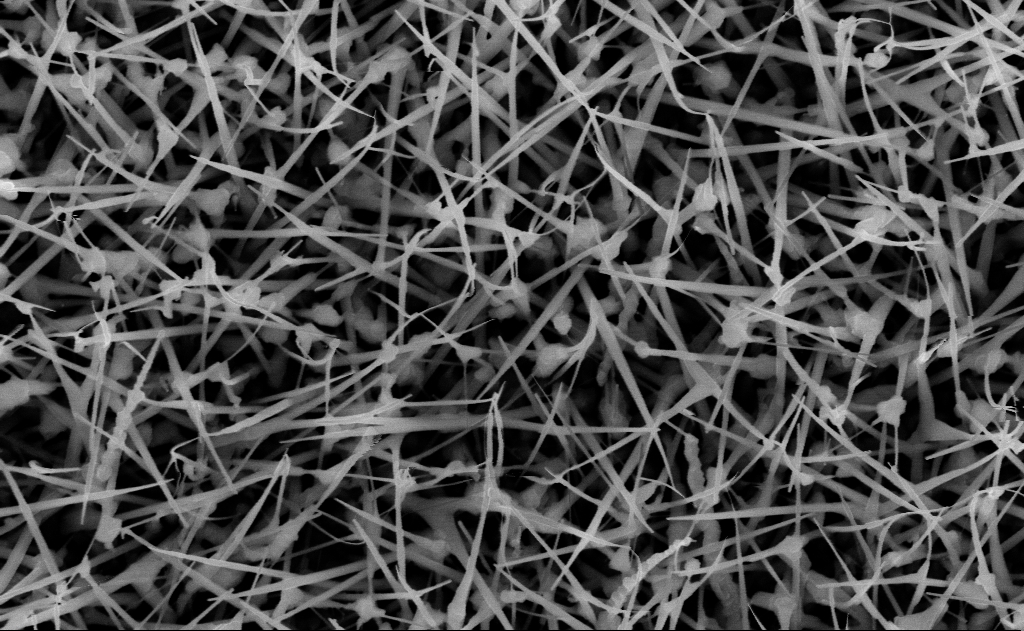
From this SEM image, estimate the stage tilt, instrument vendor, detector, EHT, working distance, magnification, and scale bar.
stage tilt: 0°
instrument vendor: Zeiss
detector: InLens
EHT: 10 kV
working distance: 15 mm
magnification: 40 K X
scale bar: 1000 nm